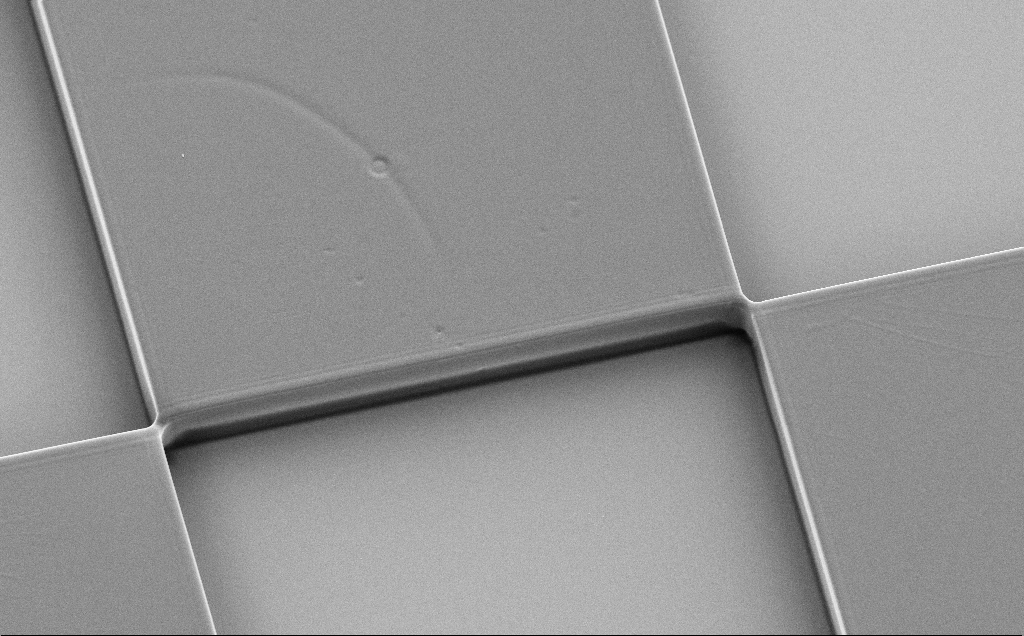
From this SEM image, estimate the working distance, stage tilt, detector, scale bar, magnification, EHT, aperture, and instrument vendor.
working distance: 11 mm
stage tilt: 30°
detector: SE2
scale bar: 10000 nm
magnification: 2.35 K X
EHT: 5 kV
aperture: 30 µm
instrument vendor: Zeiss